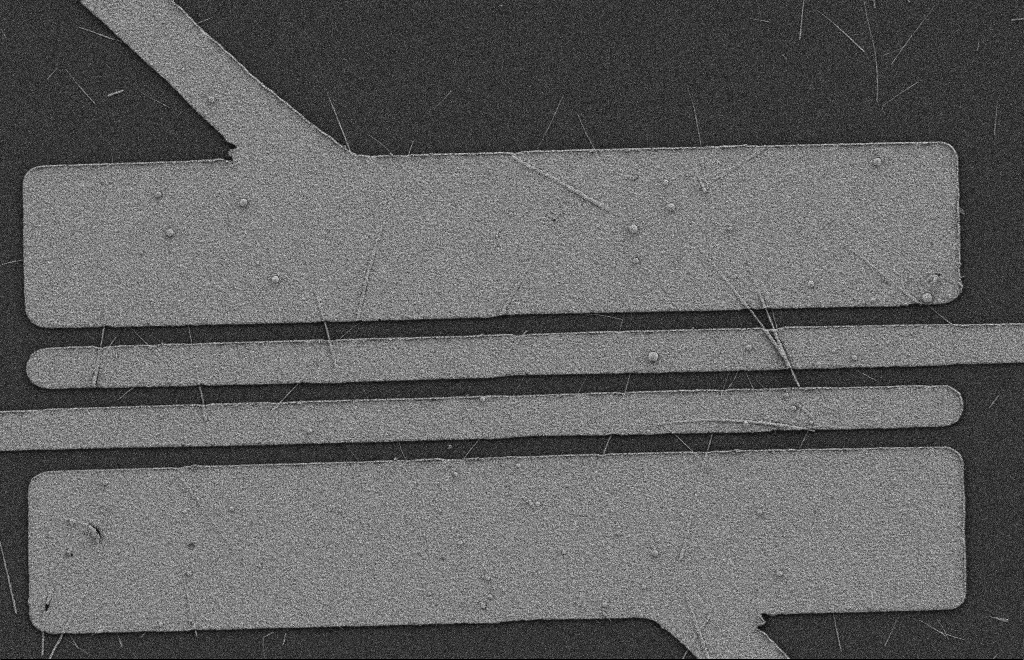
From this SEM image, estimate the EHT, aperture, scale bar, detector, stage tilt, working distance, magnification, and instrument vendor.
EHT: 2 kV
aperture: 20 µm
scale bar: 2000 nm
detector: SE2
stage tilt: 0°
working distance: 9 mm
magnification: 5.61 K X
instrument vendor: Zeiss